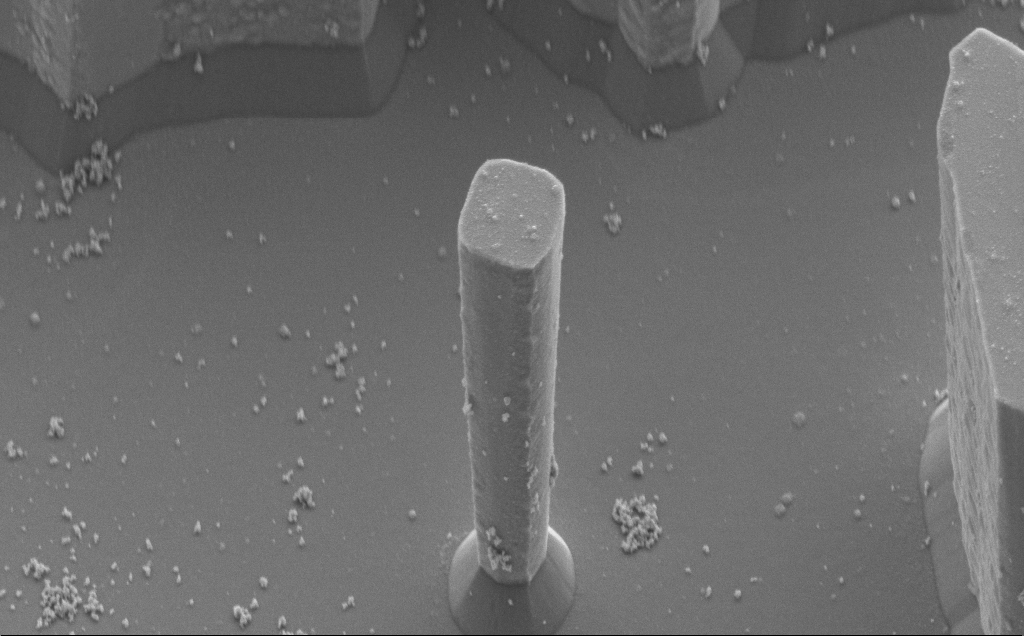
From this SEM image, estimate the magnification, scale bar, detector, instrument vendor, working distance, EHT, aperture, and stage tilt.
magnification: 6.94 K X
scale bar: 10000 nm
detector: SE2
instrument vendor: Zeiss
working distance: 10 mm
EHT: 5 kV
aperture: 30 µm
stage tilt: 46.4°